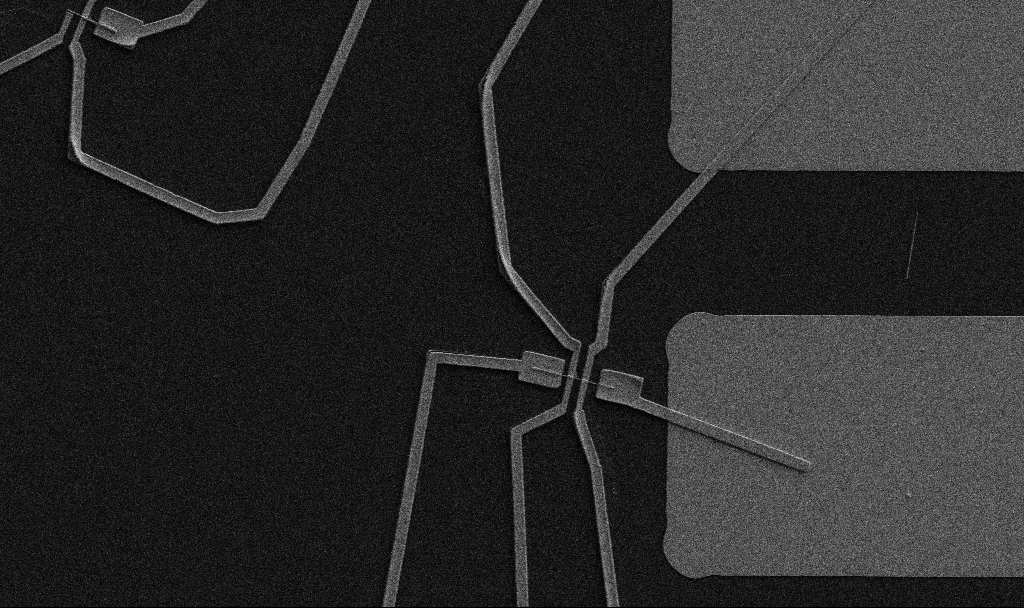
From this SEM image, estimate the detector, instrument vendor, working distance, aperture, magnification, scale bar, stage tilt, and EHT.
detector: SE2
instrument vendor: Zeiss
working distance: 10.7 mm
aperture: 30 µm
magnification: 5 K X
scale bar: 10000 nm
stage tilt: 0°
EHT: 5 kV